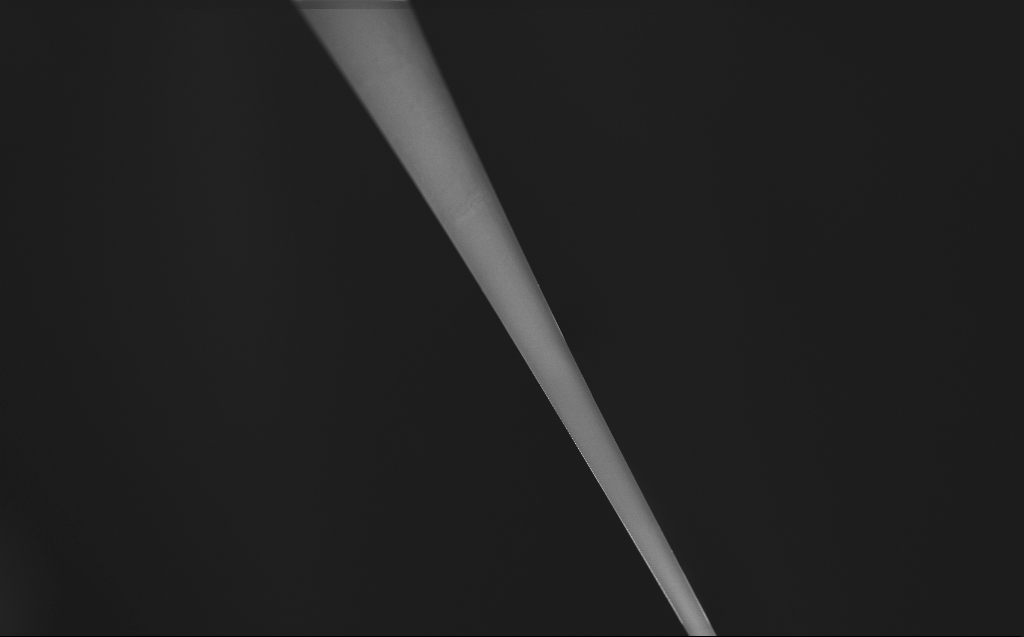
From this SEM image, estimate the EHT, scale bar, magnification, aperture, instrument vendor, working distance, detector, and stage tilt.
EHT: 0.8 kV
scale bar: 200000 nm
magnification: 0.25 K X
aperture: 30 µm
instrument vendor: Zeiss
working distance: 4 mm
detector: InLens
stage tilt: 45°